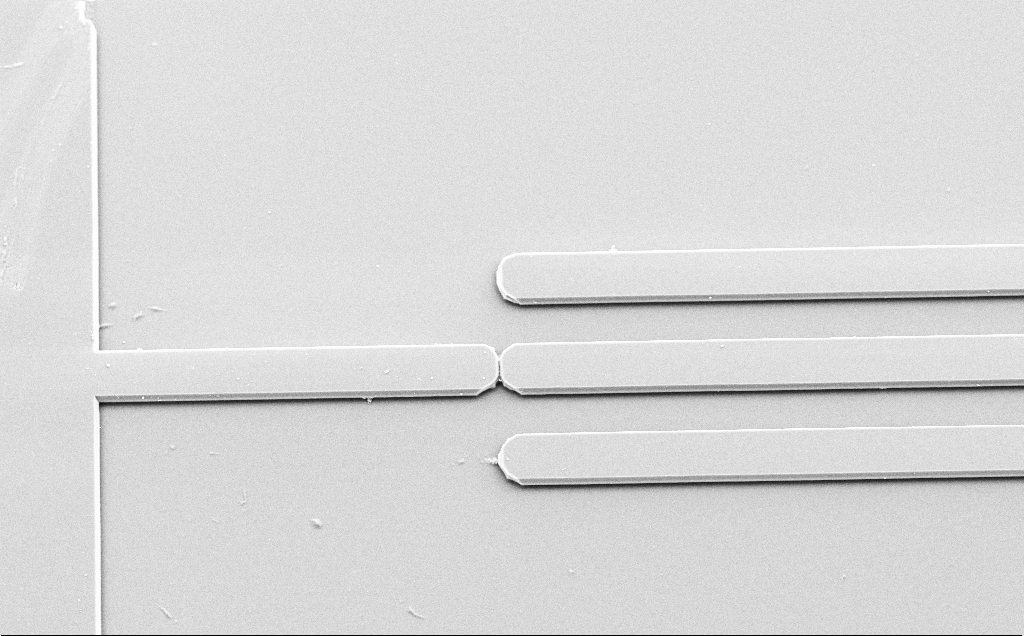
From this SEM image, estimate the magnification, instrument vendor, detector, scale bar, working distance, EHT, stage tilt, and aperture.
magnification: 0.987 K X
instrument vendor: Zeiss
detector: SE2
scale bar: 20000 nm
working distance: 10 mm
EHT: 10 kV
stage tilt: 31.9°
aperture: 30 µm